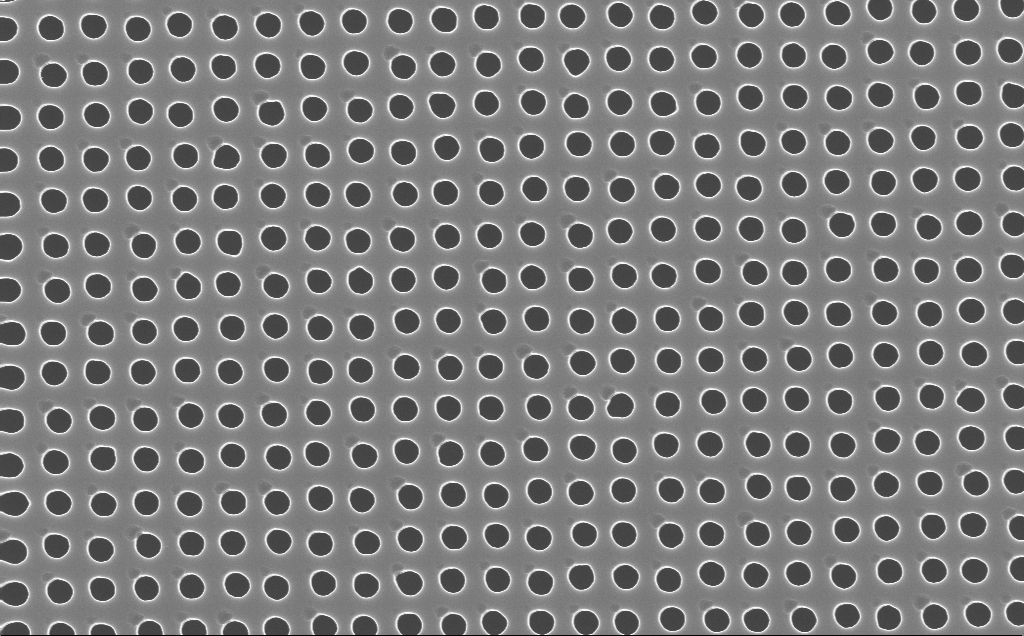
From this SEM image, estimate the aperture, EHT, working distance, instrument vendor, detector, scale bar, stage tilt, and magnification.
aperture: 30 µm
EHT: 10 kV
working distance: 4 mm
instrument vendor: Zeiss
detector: InLens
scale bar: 1000 nm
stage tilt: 0°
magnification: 40 K X